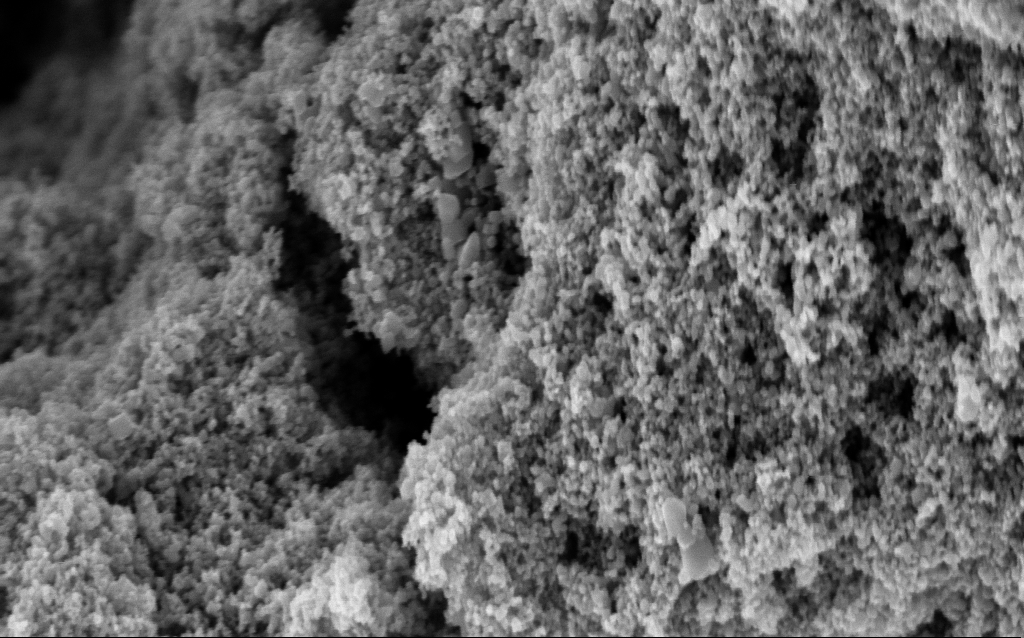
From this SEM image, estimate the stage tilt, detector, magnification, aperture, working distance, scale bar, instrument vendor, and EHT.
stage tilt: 0°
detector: InLens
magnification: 76.33 K X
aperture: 30 µm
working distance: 9.6 mm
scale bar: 200 nm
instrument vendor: Zeiss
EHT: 5 kV